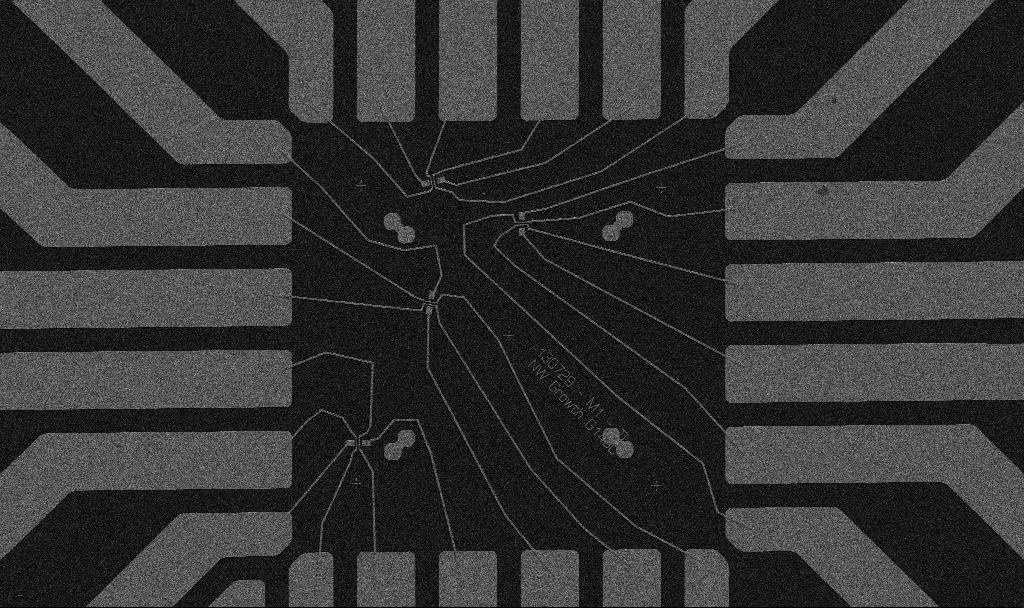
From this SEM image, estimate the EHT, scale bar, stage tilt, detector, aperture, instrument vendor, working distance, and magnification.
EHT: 5 kV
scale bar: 20000 nm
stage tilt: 0°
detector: SE2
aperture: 30 µm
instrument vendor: Zeiss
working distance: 10.7 mm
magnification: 1 K X